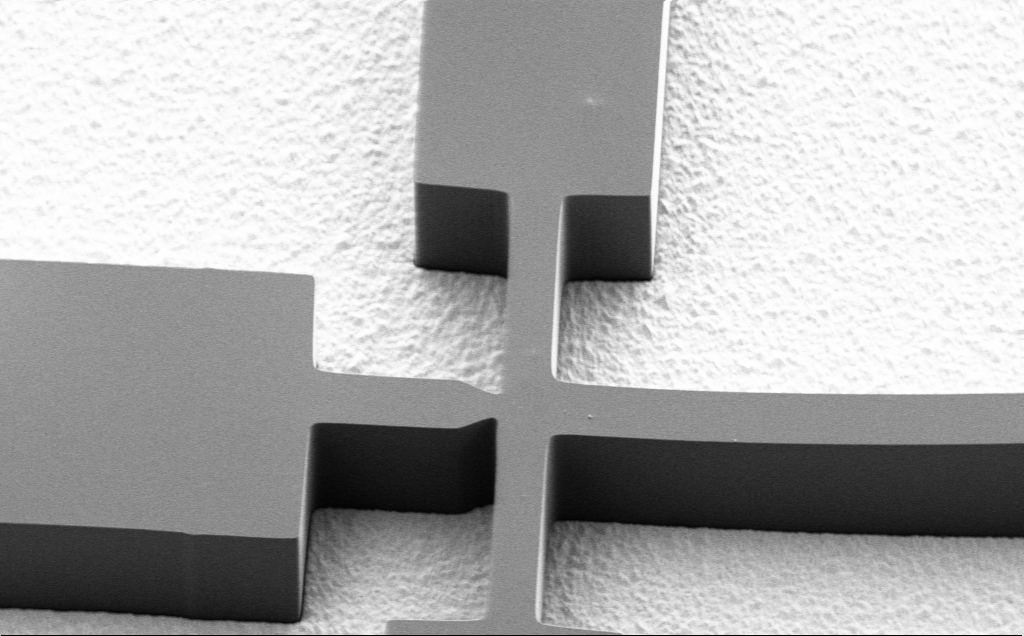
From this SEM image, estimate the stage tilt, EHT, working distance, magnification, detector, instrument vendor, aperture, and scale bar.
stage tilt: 37.9°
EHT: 1.5 kV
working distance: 8 mm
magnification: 1.78 K X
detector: SE2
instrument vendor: Zeiss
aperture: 30 µm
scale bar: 20000 nm